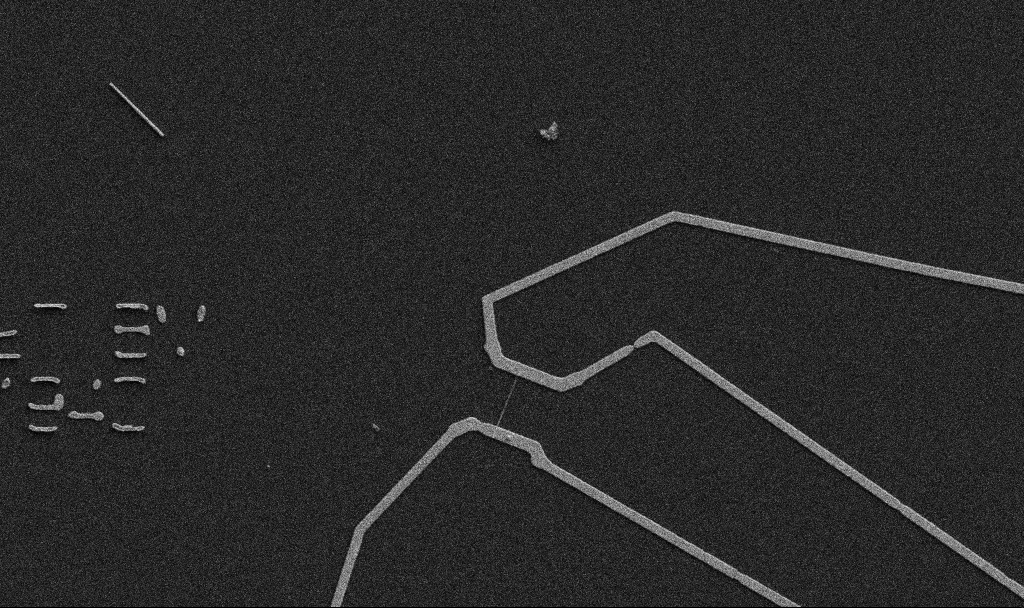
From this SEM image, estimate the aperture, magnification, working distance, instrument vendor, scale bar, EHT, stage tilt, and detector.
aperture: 30 µm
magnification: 5 K X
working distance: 10.7 mm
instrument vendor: Zeiss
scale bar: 10000 nm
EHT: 5 kV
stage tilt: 0°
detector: SE2